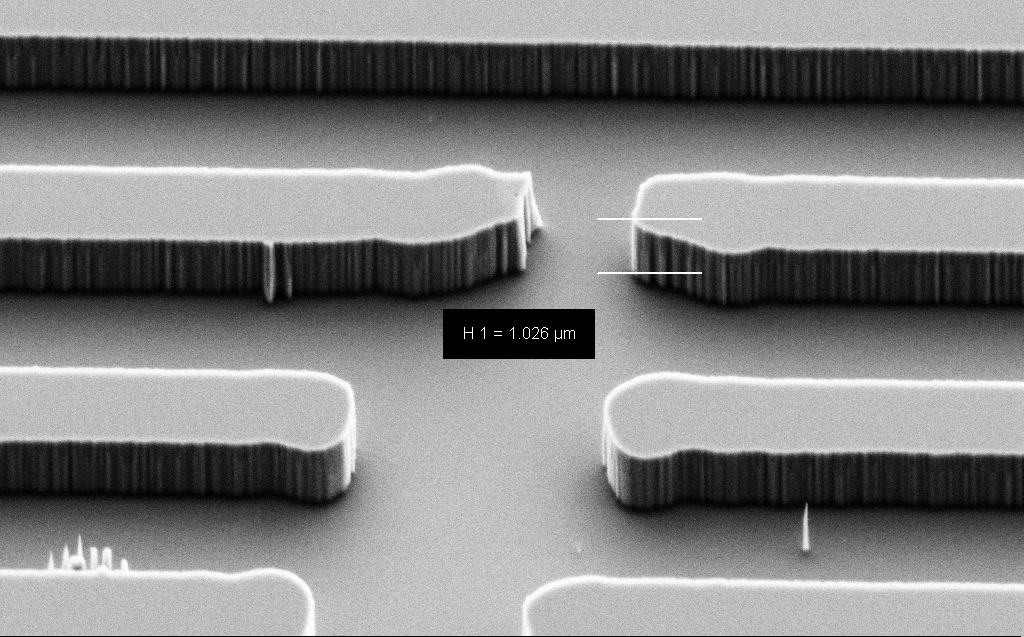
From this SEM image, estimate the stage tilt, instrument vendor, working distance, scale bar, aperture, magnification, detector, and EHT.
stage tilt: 45°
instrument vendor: Zeiss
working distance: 9 mm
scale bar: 2000 nm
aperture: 30 µm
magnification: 19.32 K X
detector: SE2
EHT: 3 kV